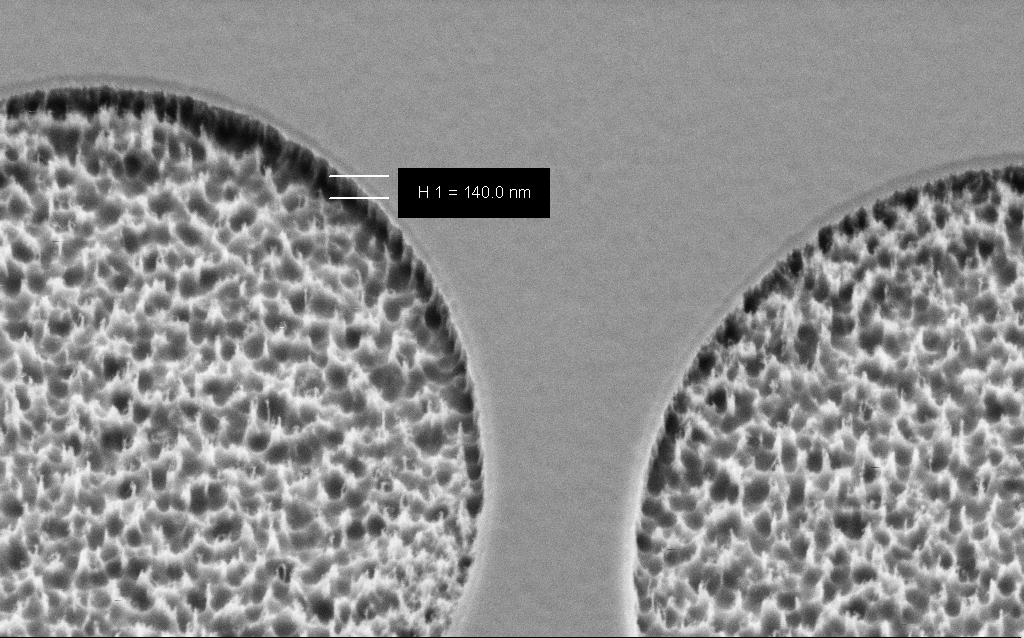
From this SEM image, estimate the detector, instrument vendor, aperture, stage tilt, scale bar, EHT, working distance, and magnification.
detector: SE2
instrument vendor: Zeiss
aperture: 30 µm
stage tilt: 45°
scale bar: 1000 nm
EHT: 3 kV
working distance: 8 mm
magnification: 57.71 K X